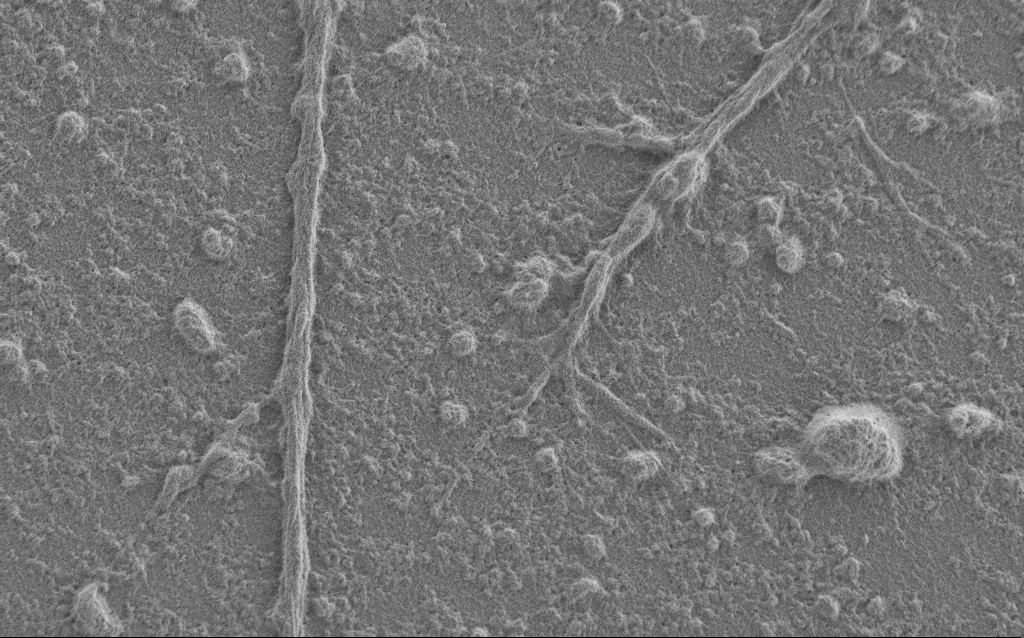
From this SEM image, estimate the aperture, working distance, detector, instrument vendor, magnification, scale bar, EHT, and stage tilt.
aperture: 30 µm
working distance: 6 mm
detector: SE2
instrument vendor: Zeiss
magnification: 7.5 K X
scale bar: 2000 nm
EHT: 1 kV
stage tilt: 0°